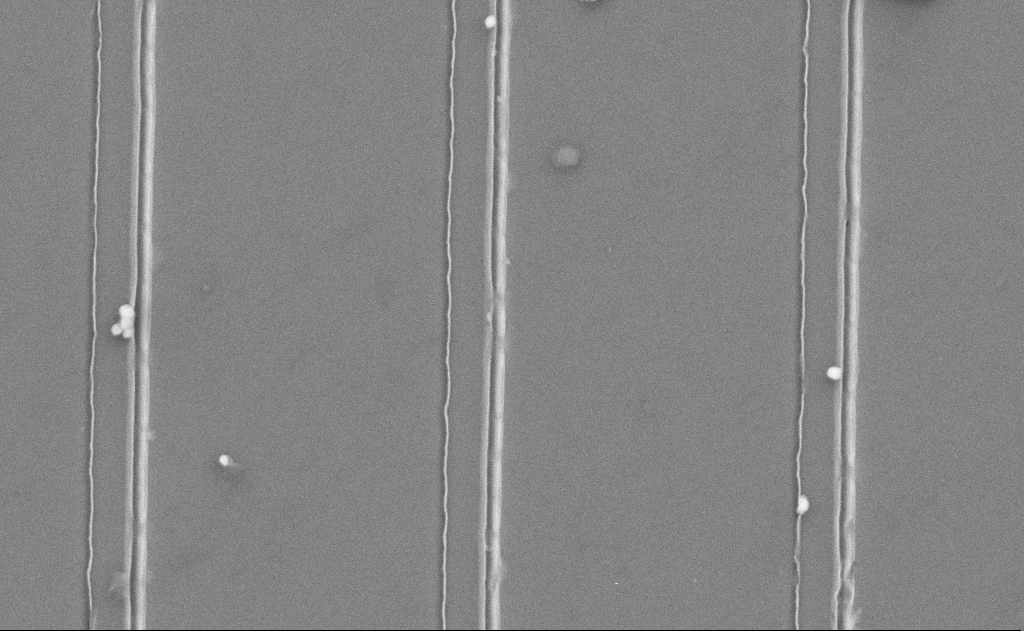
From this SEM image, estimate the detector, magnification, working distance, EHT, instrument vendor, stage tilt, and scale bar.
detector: SE2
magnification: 32.27 K X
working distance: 12 mm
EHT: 5 kV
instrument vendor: Zeiss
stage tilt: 0°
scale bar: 1000 nm